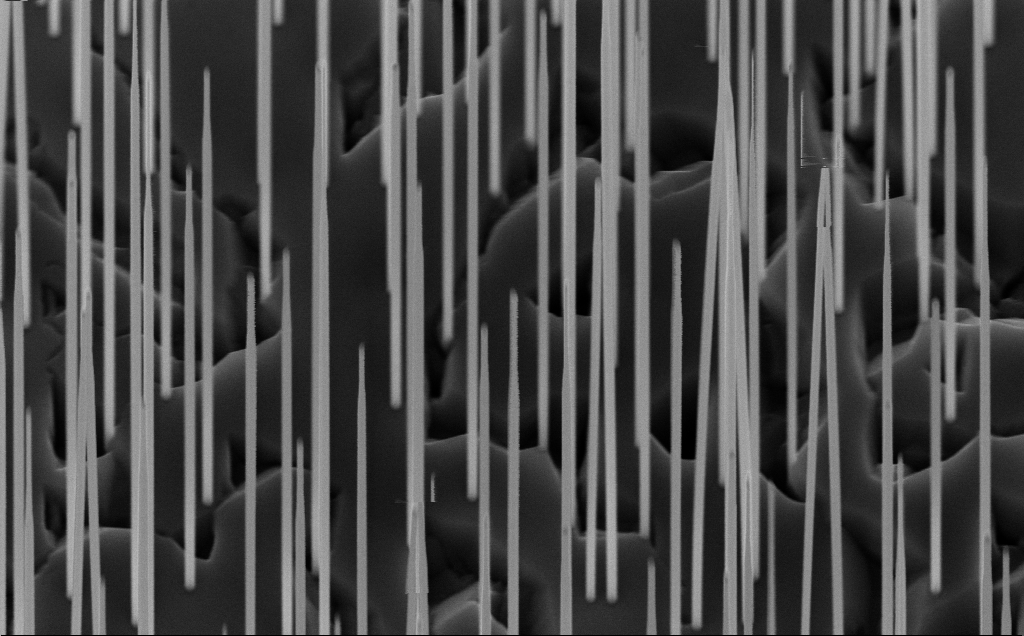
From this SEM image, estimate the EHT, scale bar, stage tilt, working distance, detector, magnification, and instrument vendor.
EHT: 10 kV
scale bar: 1000 nm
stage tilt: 45°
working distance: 7 mm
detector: InLens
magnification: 39.98 K X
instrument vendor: Zeiss